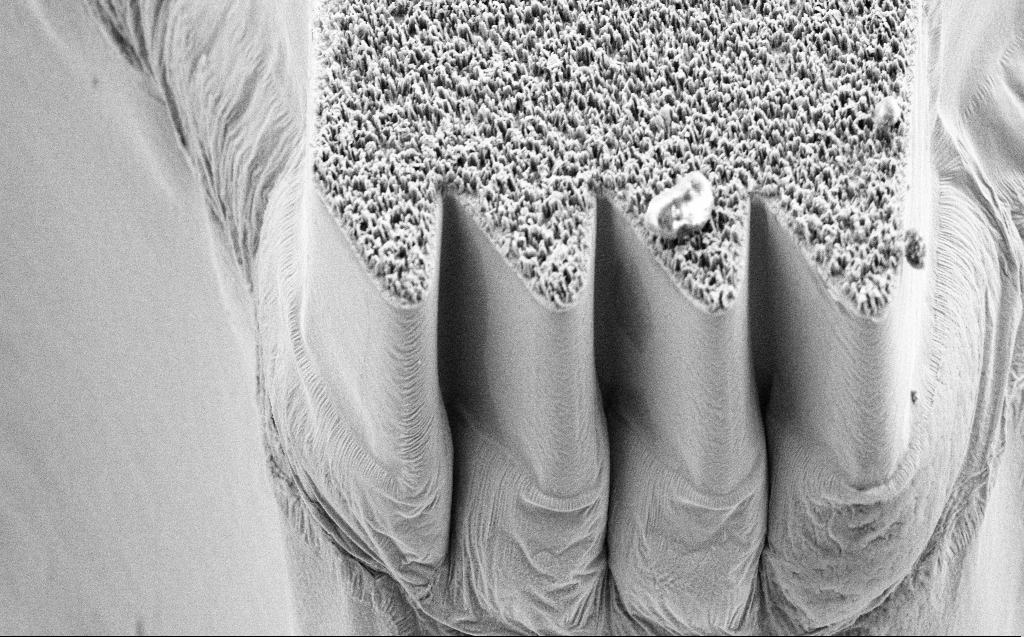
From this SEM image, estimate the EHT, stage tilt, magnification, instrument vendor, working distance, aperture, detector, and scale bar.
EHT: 5 kV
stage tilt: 45°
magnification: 4.99 K X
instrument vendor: Zeiss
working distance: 9 mm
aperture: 30 µm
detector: SE2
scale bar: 10000 nm